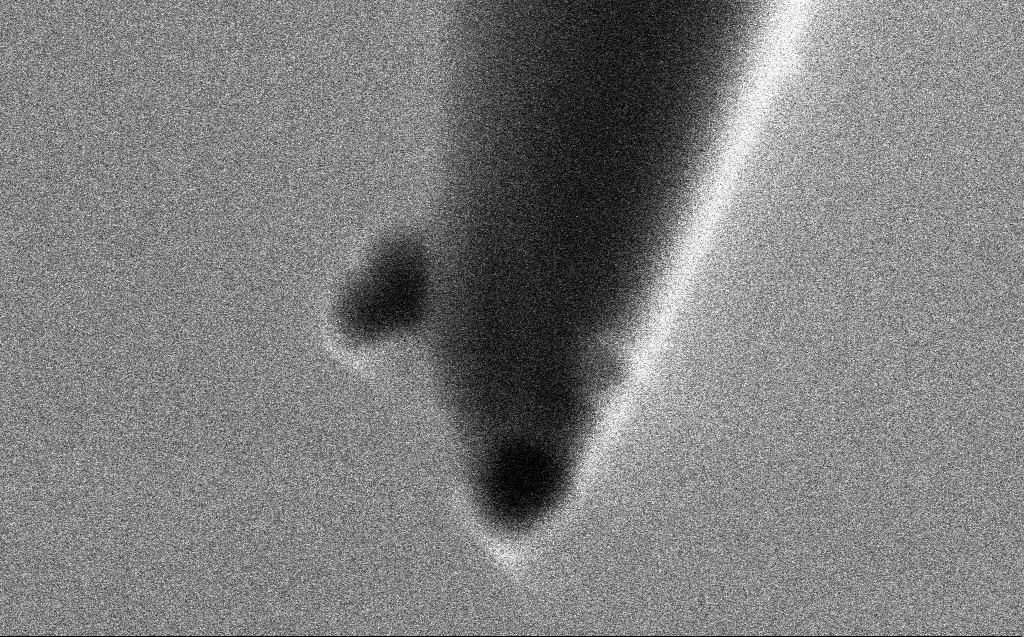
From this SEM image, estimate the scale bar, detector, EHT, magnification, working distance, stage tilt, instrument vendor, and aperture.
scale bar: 100 nm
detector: SE2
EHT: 1 kV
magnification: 360.78 K X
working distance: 3 mm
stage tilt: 45.1°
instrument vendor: Zeiss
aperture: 30 µm